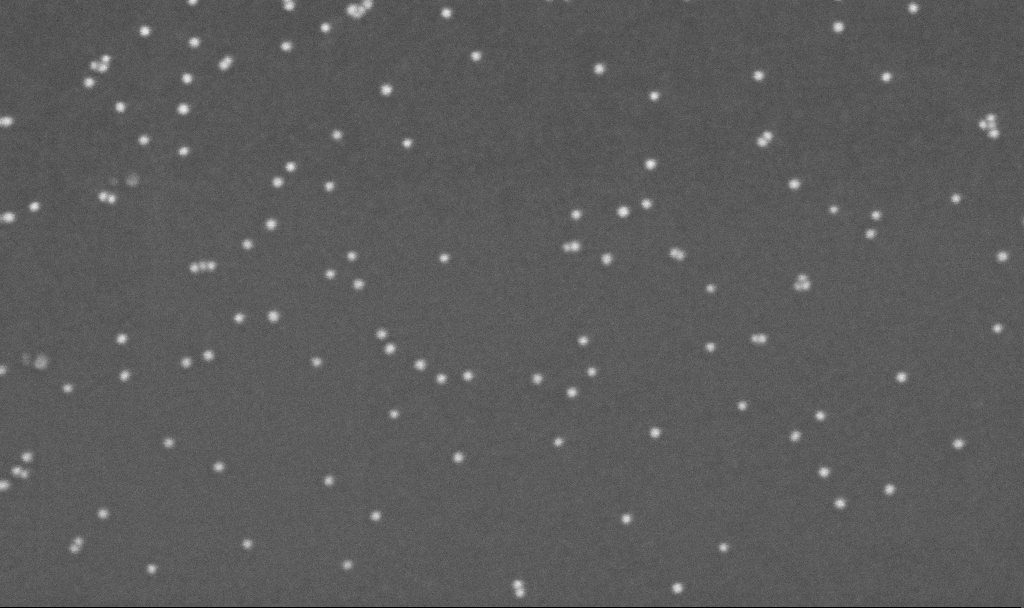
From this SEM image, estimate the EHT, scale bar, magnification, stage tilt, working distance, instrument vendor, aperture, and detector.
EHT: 10 kV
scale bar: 200 nm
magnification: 300 K X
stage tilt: -0°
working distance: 3.3 mm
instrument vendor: Zeiss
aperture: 30 µm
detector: InLens